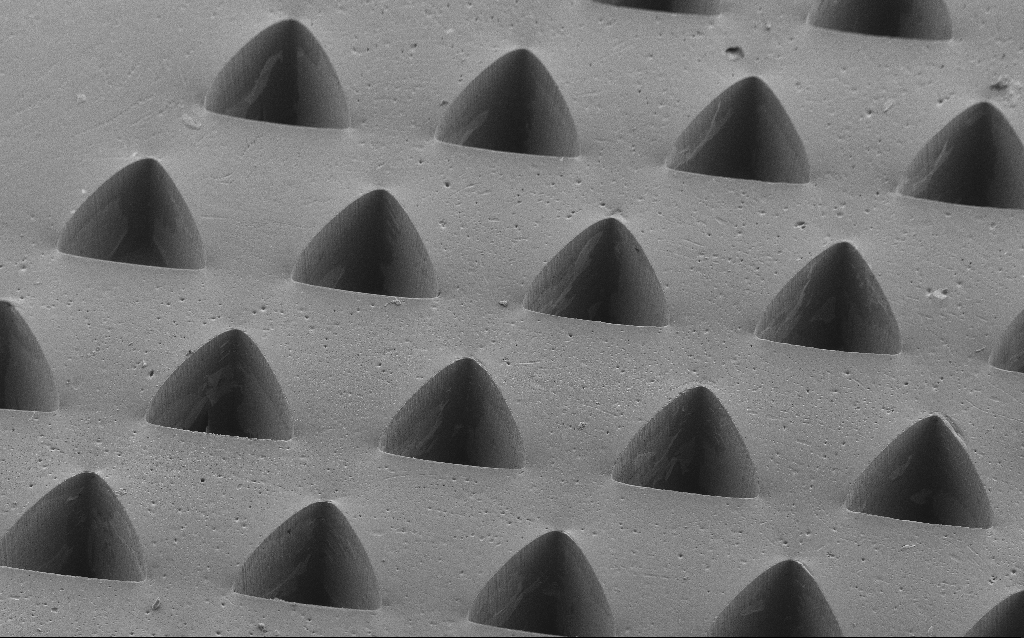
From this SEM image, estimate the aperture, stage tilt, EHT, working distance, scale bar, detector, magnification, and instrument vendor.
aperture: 30 µm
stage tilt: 35°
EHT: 5 kV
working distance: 8 mm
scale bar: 100000 nm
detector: SE2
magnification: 0.195 K X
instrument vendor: Zeiss